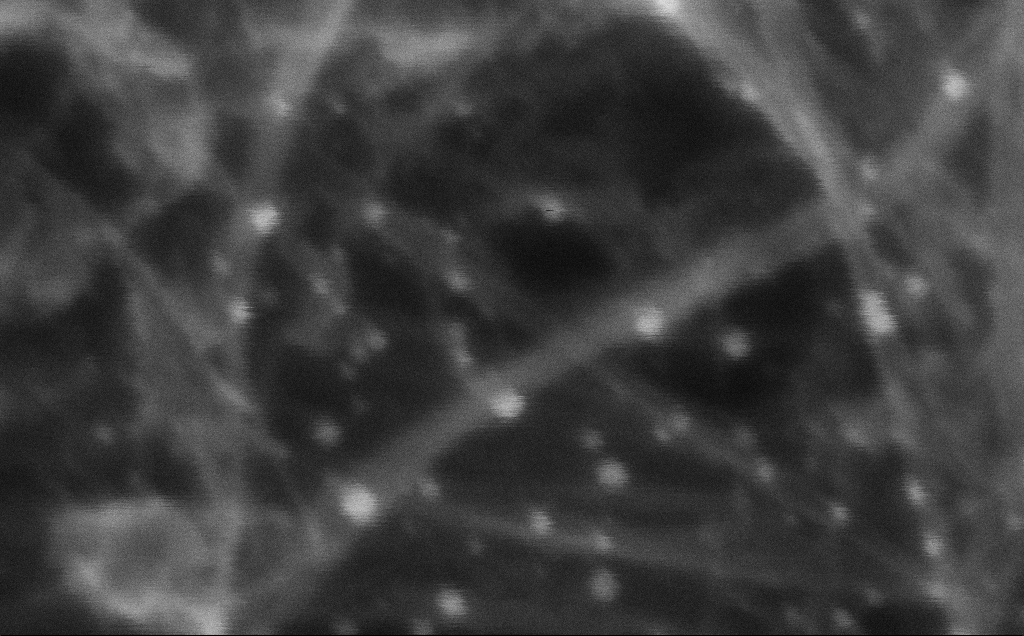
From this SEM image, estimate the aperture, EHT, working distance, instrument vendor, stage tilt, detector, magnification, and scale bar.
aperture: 30 µm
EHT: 10 kV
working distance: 3 mm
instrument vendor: Zeiss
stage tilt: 0°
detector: InLens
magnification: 978.84 K X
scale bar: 20 nm